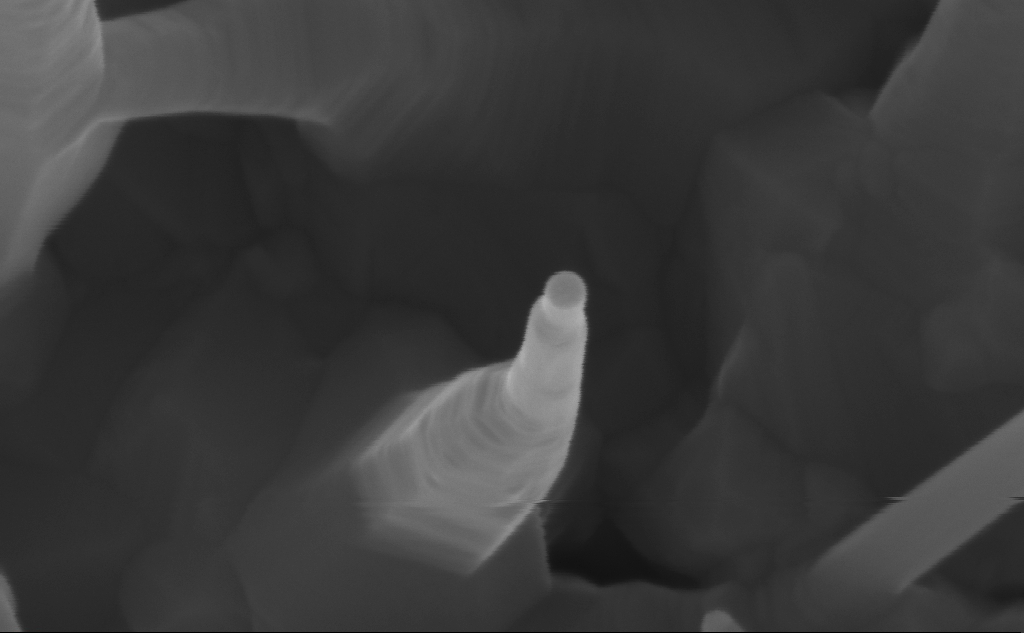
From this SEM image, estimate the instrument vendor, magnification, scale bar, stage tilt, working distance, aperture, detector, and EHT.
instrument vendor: Zeiss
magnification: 354.85 K X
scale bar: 200 nm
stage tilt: -0°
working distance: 7 mm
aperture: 30 µm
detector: InLens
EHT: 10 kV